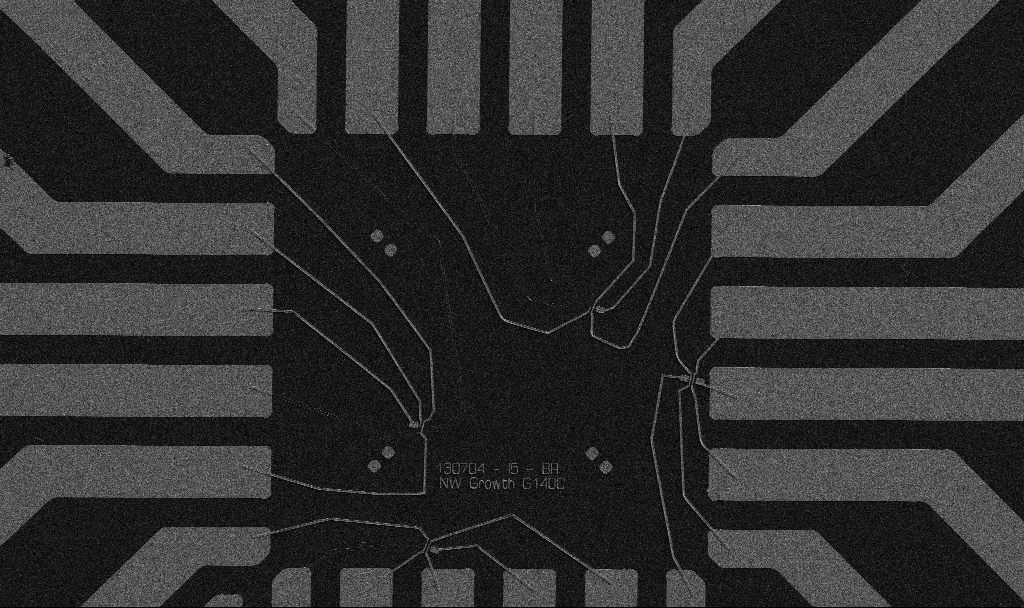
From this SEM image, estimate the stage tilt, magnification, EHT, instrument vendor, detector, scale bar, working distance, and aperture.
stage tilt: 0°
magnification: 1 K X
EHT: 5 kV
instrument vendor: Zeiss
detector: SE2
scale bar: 20000 nm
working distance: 10.7 mm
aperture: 30 µm